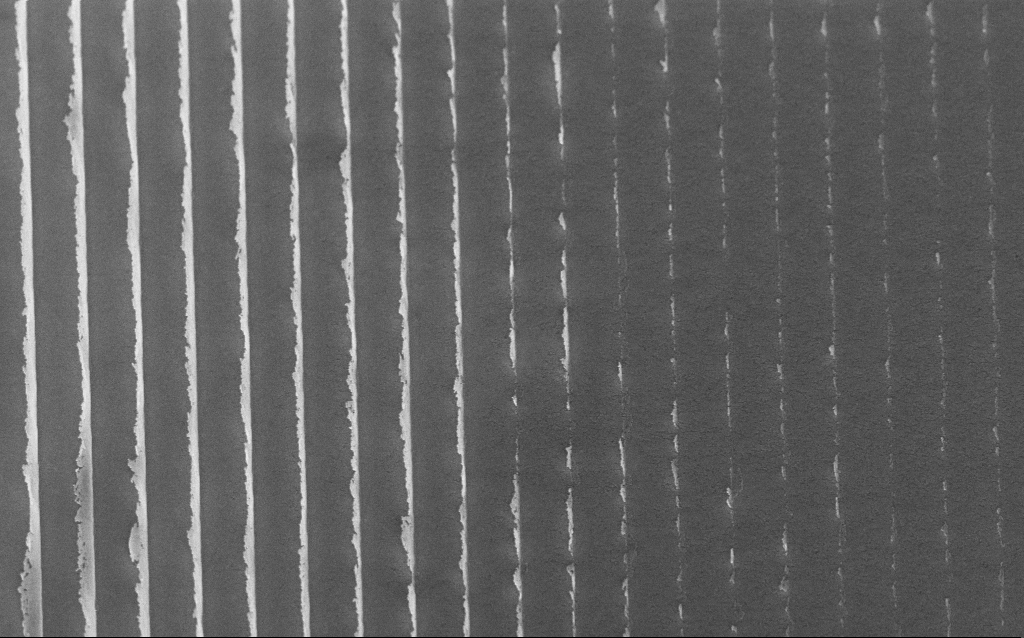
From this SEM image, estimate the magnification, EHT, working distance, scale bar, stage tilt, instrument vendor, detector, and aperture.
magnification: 39.56 K X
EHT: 10 kV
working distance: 7 mm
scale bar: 1000 nm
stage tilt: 45°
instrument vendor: Zeiss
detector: InLens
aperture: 30 µm